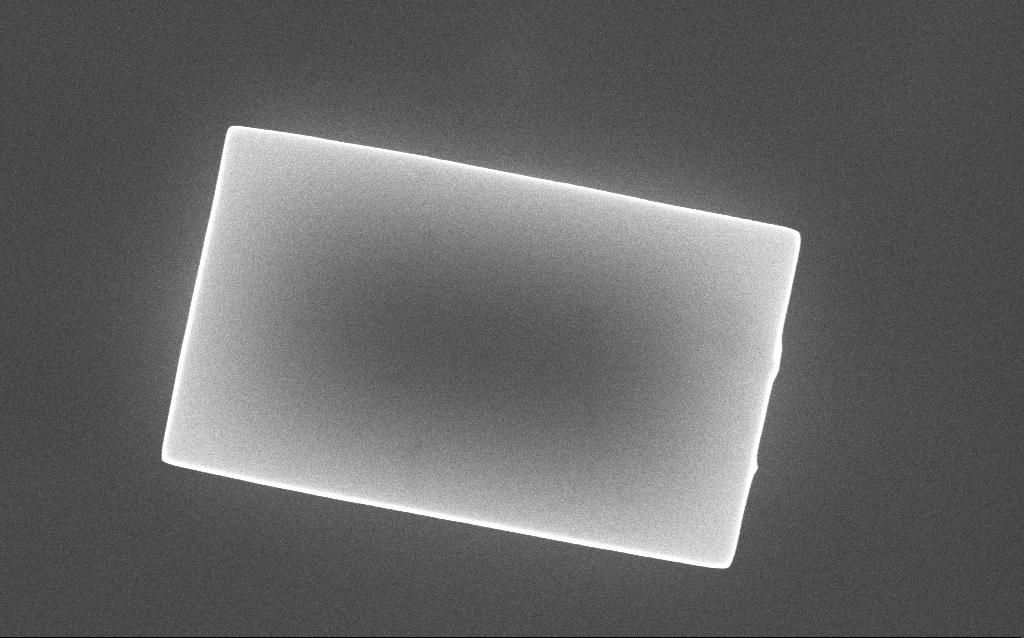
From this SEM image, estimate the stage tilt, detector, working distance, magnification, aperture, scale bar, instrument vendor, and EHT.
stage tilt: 0°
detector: InLens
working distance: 5.4 mm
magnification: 82.85 K X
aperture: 30 µm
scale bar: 200 nm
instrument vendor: Zeiss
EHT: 10 kV